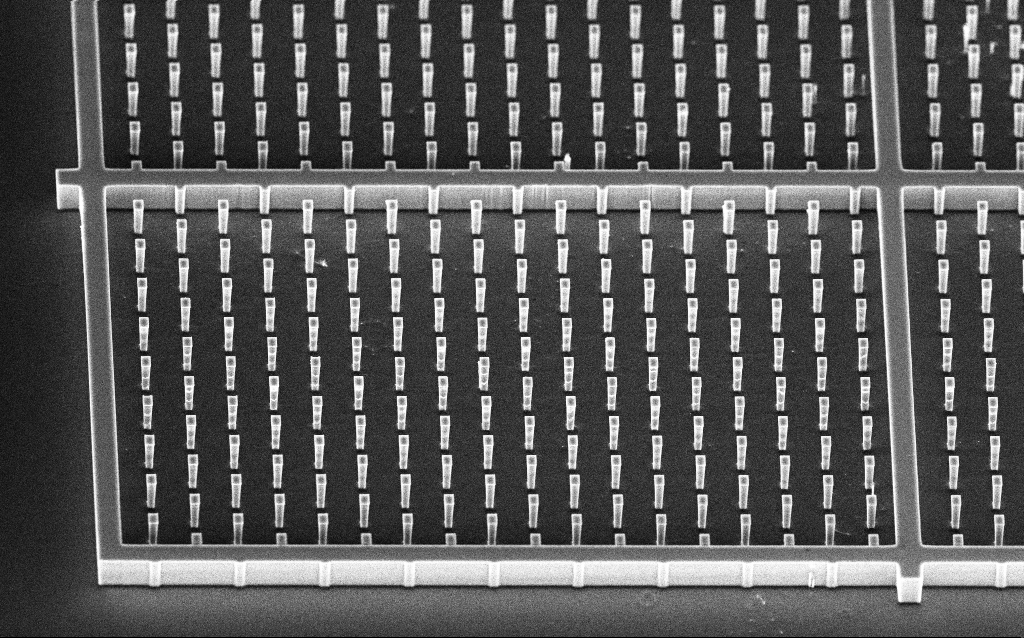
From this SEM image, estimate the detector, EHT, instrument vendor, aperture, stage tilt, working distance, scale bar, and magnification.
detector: InLens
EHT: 10 kV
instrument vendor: Zeiss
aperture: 30 µm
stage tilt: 45°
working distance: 4.8 mm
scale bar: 10000 nm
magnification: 1.72 K X